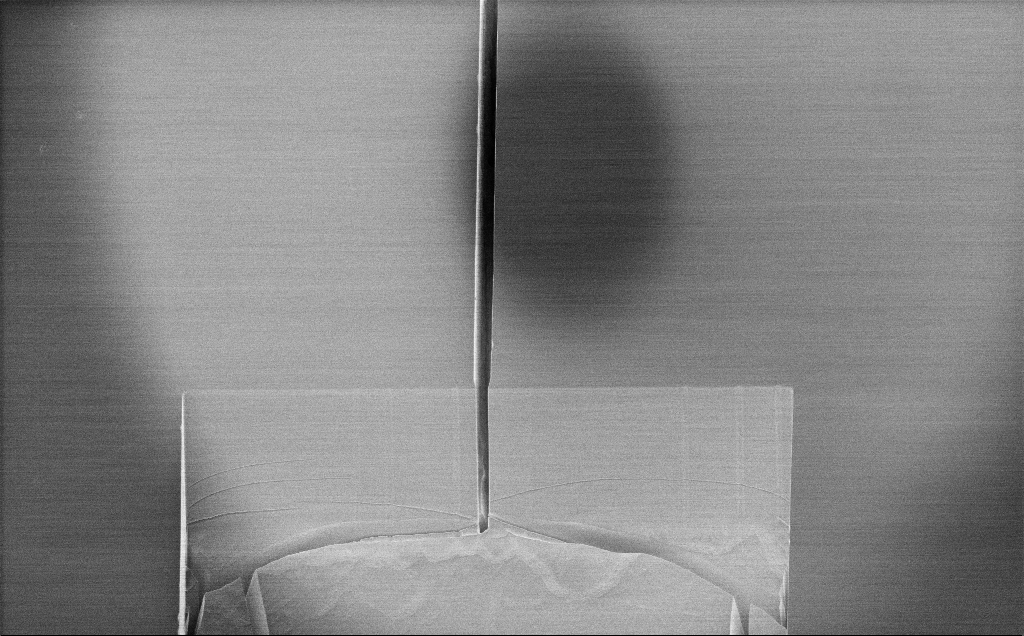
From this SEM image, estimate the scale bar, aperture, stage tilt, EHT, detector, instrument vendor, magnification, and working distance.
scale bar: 100000 nm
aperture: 30 µm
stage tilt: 45°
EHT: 1.2 kV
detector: InLens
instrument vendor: Zeiss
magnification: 0.56 K X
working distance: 6 mm